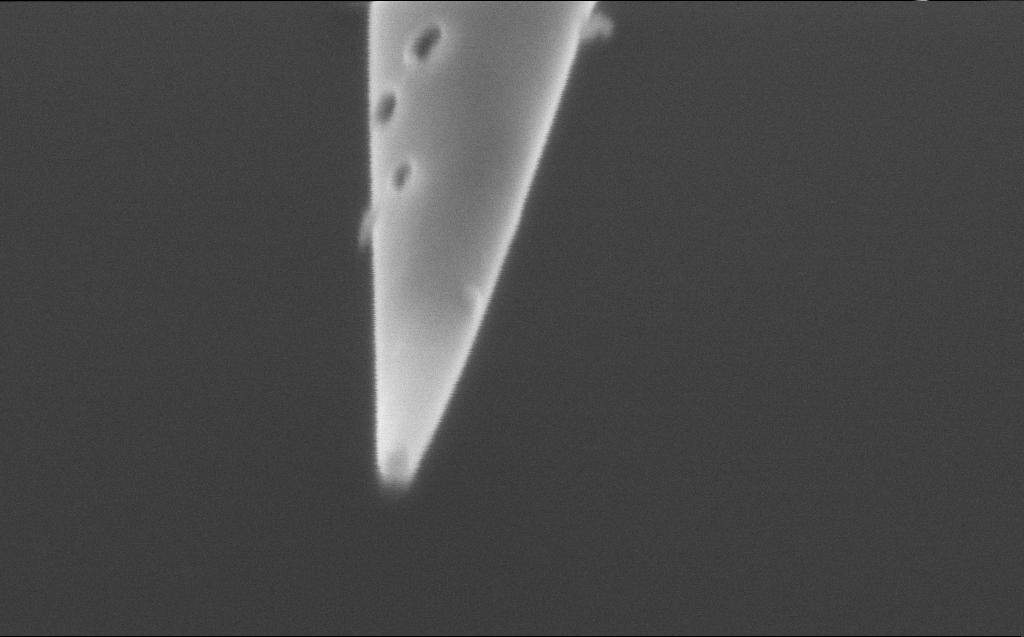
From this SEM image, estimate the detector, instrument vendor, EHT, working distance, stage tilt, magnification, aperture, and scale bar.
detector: InLens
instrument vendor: Zeiss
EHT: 1.5 kV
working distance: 3 mm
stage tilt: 45.1°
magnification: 207.83 K X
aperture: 20 µm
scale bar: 100 nm